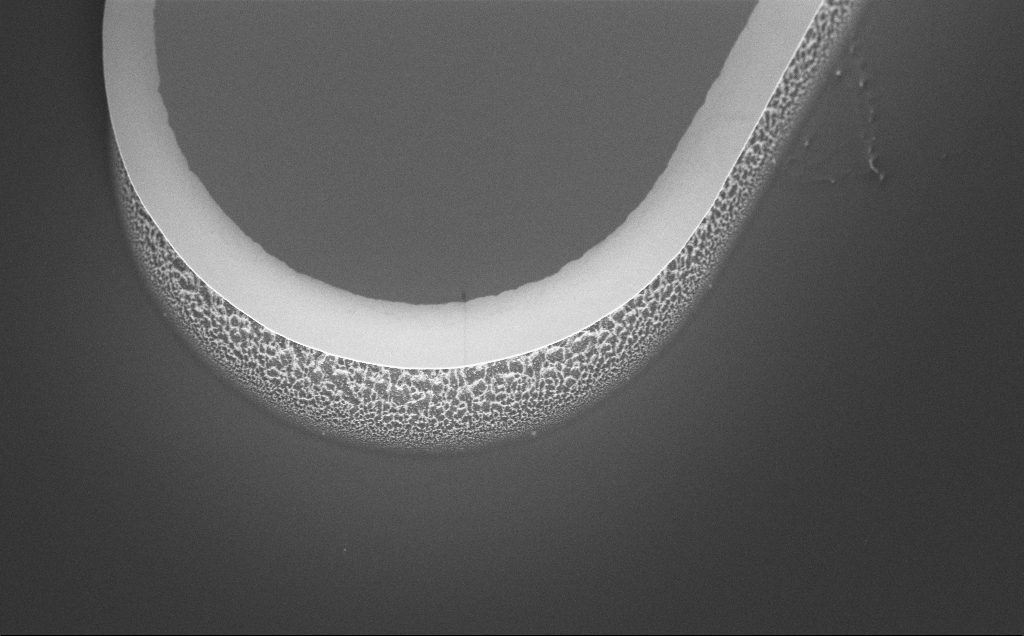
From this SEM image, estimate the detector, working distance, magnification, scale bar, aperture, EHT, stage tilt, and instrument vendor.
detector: InLens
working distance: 8 mm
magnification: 3.01 K X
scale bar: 20000 nm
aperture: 30 µm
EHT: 5 kV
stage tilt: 45°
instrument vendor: Zeiss